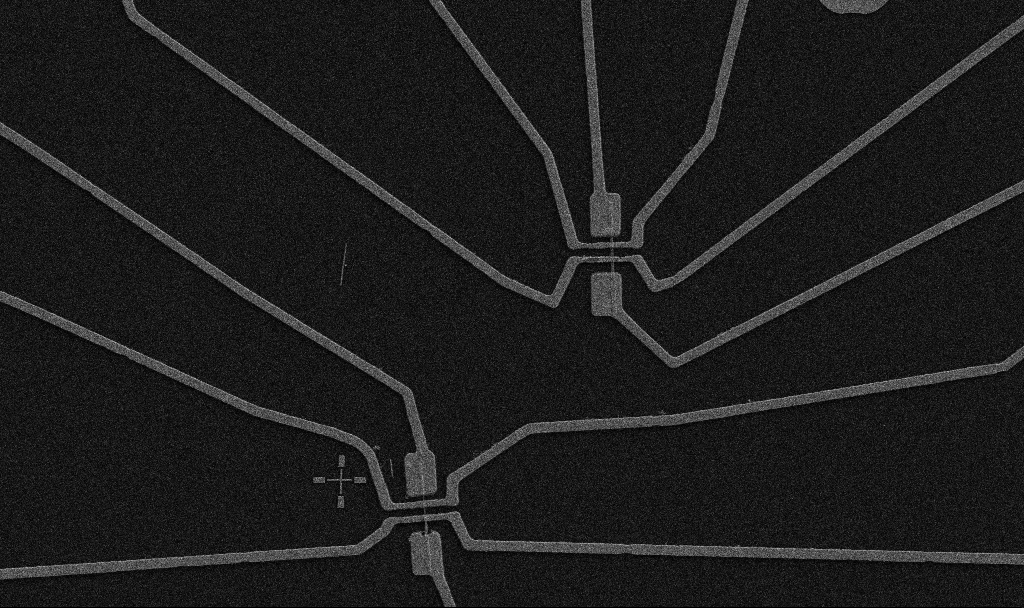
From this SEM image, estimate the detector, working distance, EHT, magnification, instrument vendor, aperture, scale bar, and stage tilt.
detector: SE2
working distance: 10.7 mm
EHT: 5 kV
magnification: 5 K X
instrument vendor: Zeiss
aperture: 30 µm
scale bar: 10000 nm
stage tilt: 0°